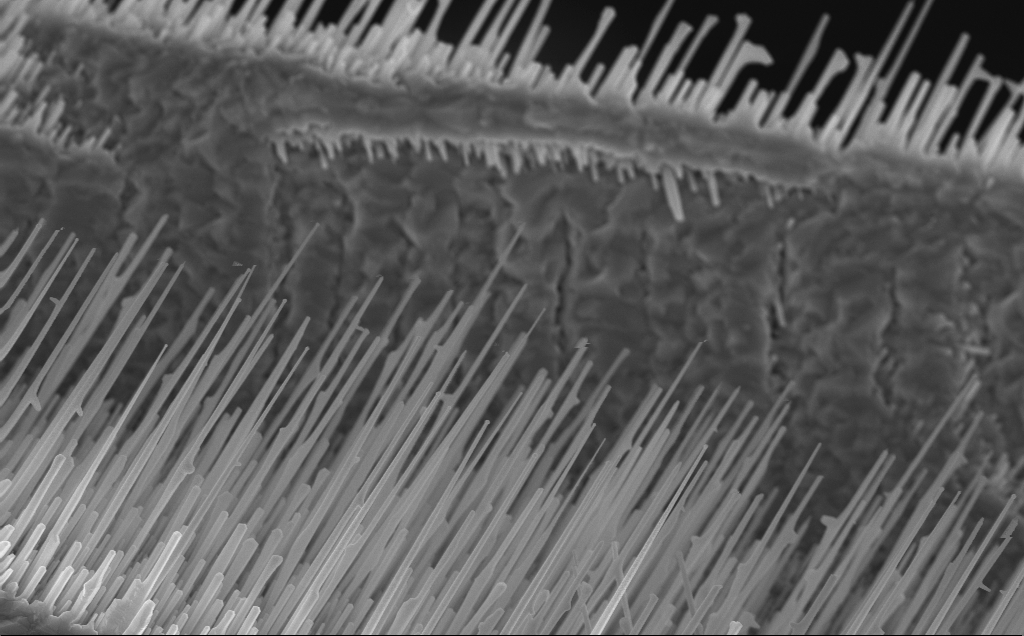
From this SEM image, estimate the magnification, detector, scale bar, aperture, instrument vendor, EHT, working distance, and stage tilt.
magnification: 16.92 K X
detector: InLens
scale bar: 2000 nm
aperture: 30 µm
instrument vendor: Zeiss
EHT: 10 kV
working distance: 7 mm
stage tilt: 0°